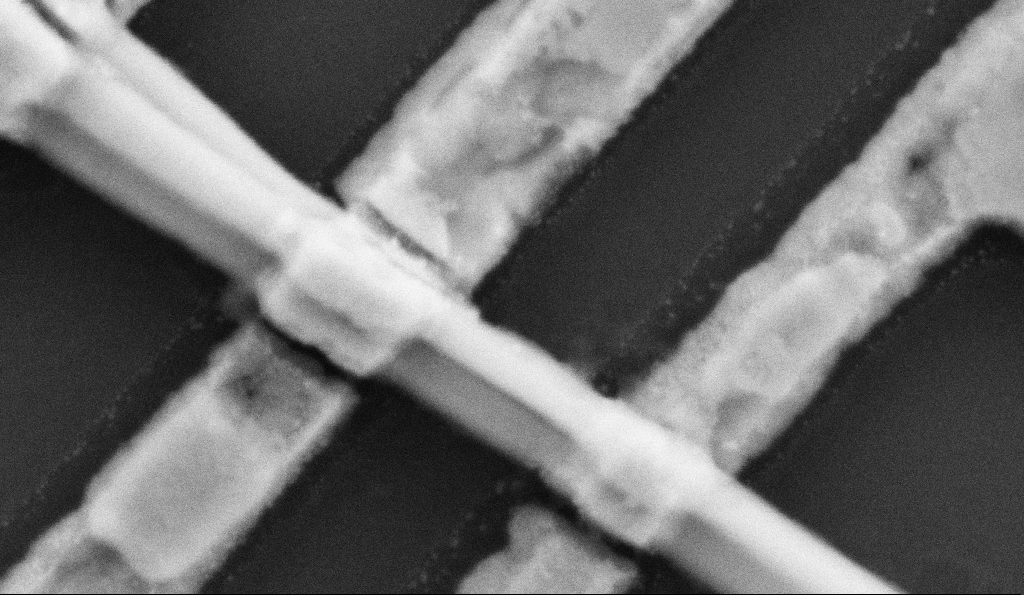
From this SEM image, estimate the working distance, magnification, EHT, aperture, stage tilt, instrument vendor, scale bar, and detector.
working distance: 8.5 mm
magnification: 200 K X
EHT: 5 kV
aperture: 30 µm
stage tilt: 0°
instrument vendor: Zeiss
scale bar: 100 nm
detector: SE2